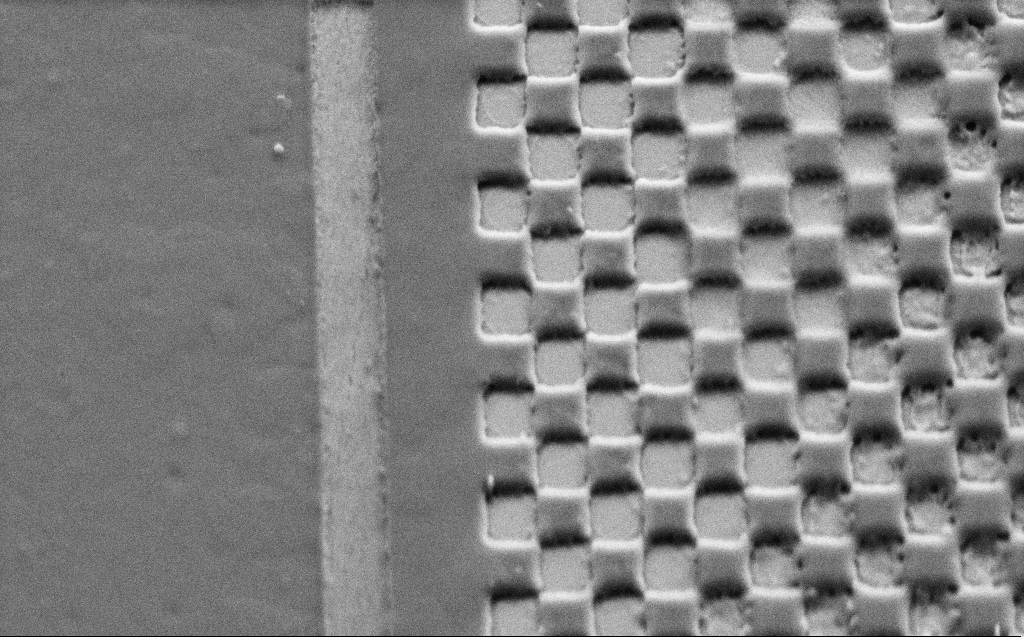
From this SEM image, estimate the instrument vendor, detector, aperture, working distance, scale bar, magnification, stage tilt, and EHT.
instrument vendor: Zeiss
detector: SE2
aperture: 30 µm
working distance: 6 mm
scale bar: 2000 nm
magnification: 19.31 K X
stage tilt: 45°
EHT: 3 kV